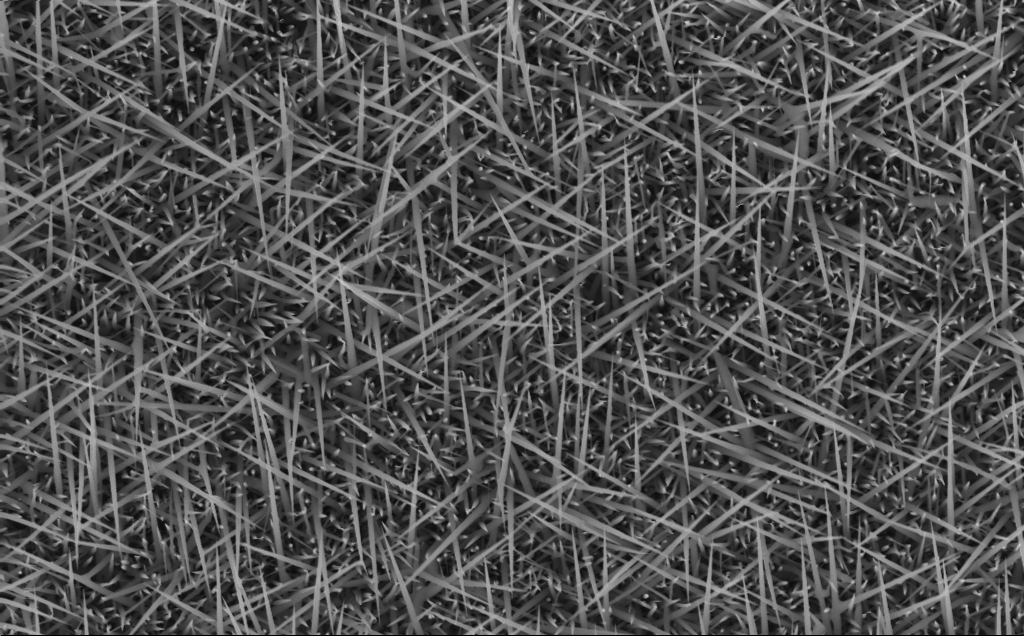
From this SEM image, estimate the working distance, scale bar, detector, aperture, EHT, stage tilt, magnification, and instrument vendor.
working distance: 6 mm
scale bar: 1000 nm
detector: InLens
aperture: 30 µm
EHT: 10 kV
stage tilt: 0°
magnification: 40 K X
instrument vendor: Zeiss